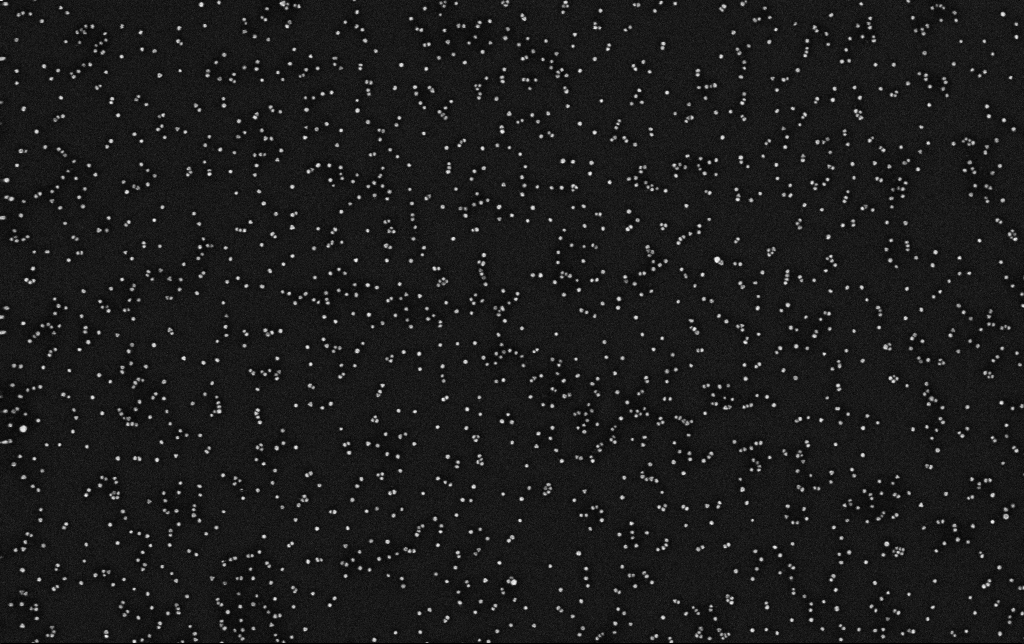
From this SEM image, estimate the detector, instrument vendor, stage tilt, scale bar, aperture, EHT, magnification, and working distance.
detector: InLens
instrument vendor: Zeiss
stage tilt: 0°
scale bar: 200 nm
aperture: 30 µm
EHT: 10 kV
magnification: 100 K X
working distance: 3.3 mm